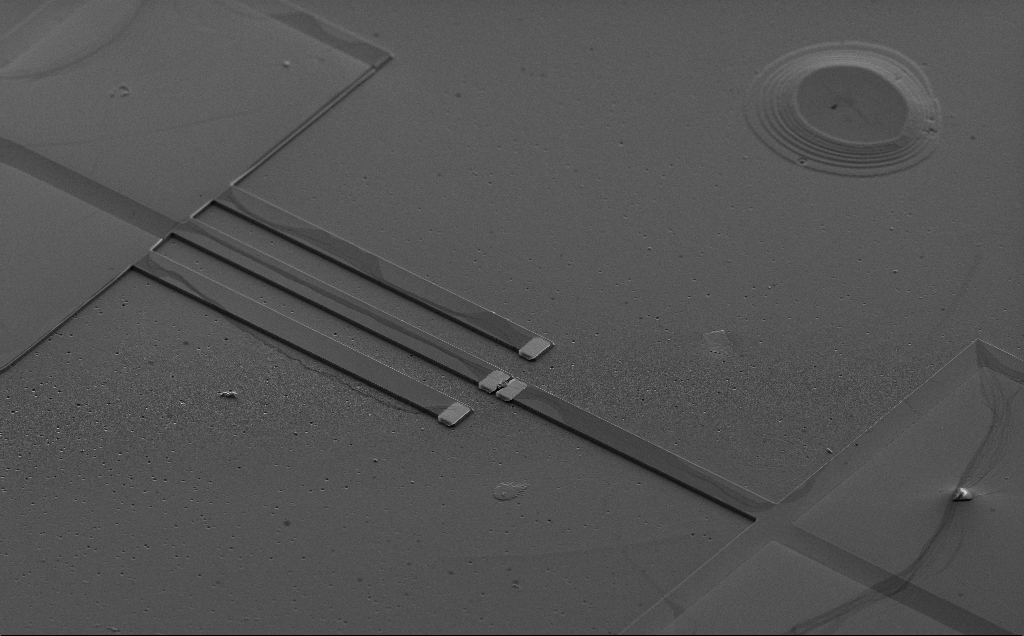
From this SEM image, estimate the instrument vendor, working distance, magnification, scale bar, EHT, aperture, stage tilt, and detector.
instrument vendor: Zeiss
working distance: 10 mm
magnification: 0.605 K X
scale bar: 100000 nm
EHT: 5 kV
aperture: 30 µm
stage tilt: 50°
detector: SE2